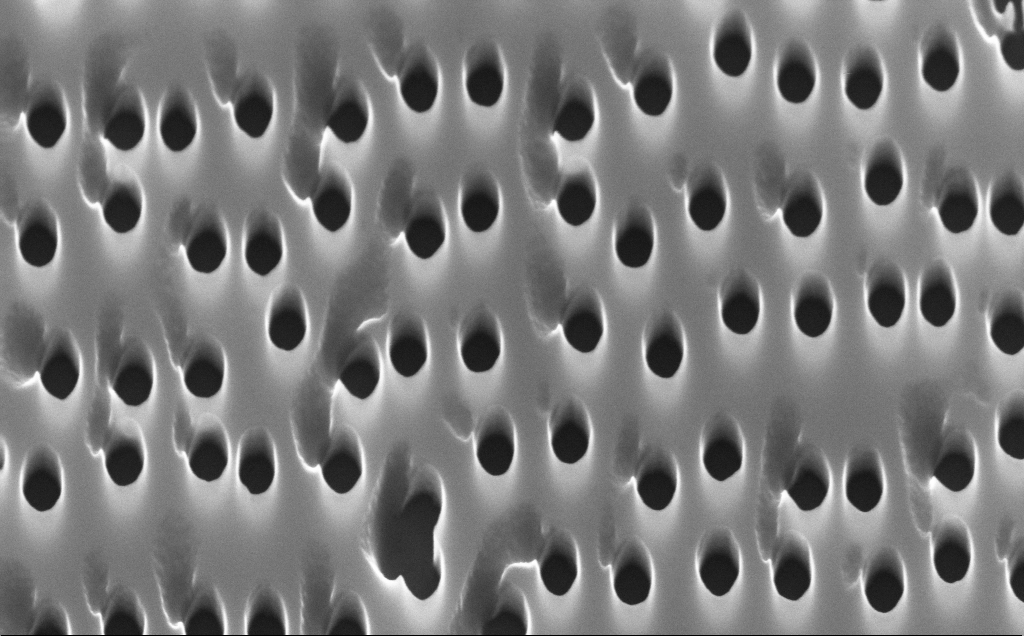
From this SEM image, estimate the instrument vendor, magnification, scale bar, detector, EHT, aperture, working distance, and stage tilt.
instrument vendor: Zeiss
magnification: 69.19 K X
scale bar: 1000 nm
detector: InLens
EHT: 10 kV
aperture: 30 µm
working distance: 4 mm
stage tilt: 30°